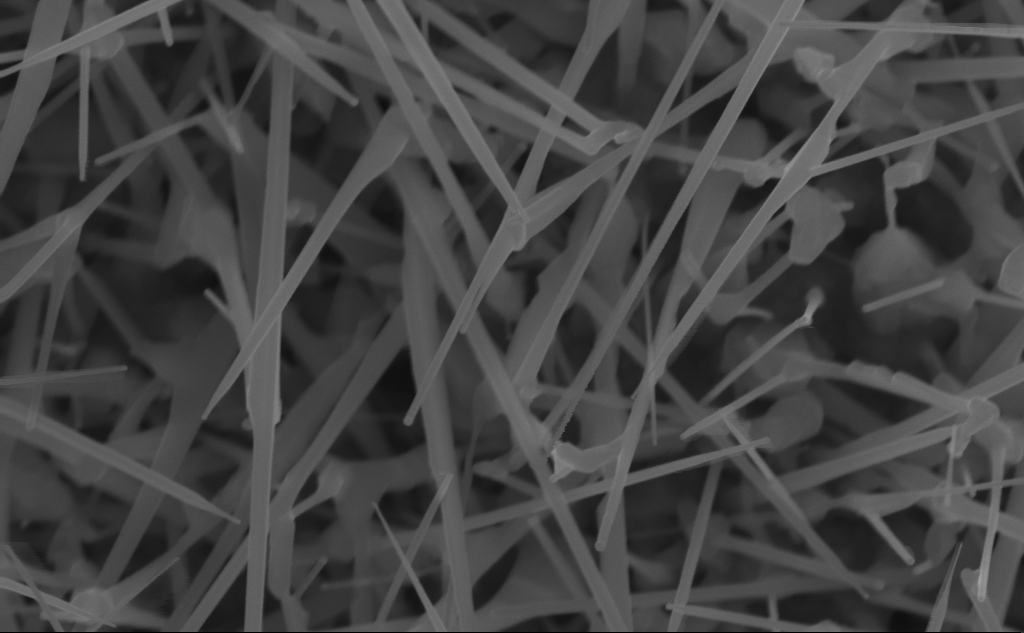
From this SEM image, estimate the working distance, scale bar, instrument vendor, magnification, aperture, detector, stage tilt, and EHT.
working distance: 6 mm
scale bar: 200 nm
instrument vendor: Zeiss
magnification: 90.32 K X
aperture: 30 µm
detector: InLens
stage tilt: -0.2°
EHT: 10 kV